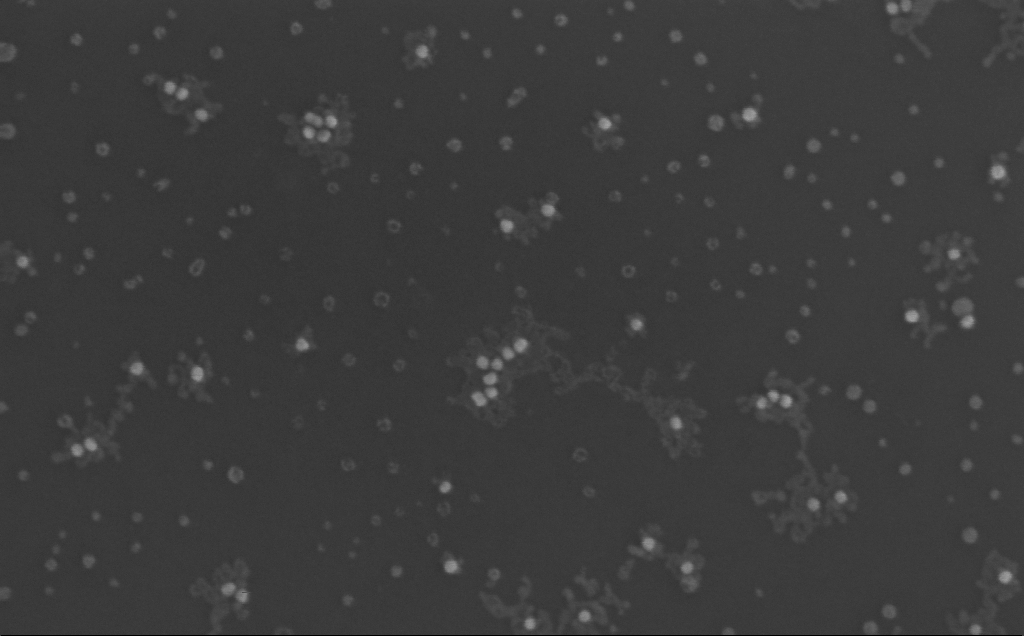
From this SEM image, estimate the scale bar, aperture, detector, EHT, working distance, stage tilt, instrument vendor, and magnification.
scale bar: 200 nm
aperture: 30 µm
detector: InLens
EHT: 10 kV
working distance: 3 mm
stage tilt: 0°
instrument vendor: Zeiss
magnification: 297.46 K X